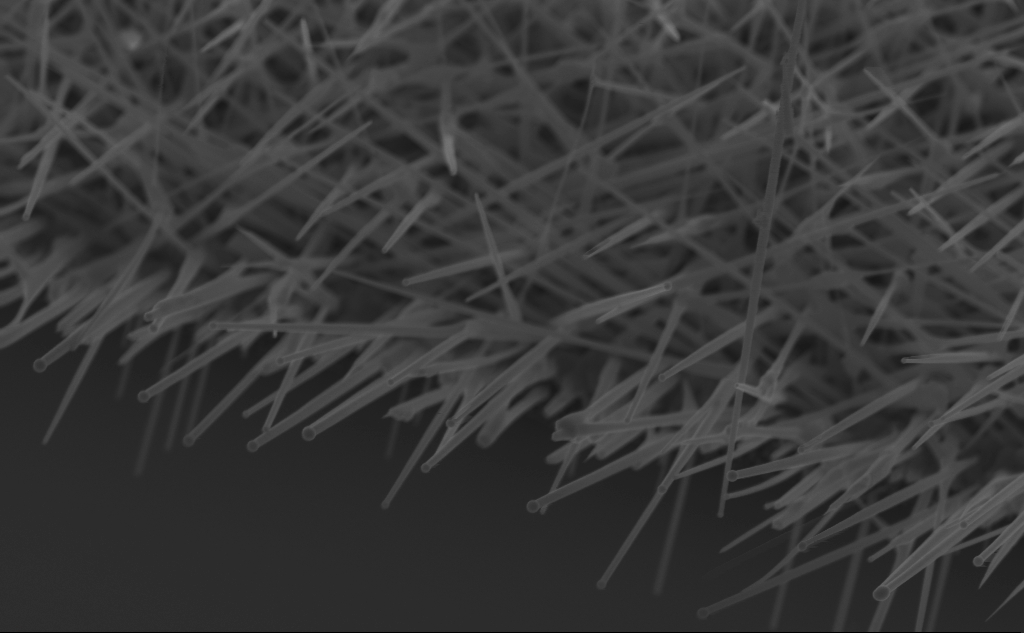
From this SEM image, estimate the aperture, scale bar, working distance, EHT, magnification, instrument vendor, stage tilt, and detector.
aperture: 30 µm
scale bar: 1000 nm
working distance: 5 mm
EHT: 10 kV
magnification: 37.89 K X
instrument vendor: Zeiss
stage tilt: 45°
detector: InLens